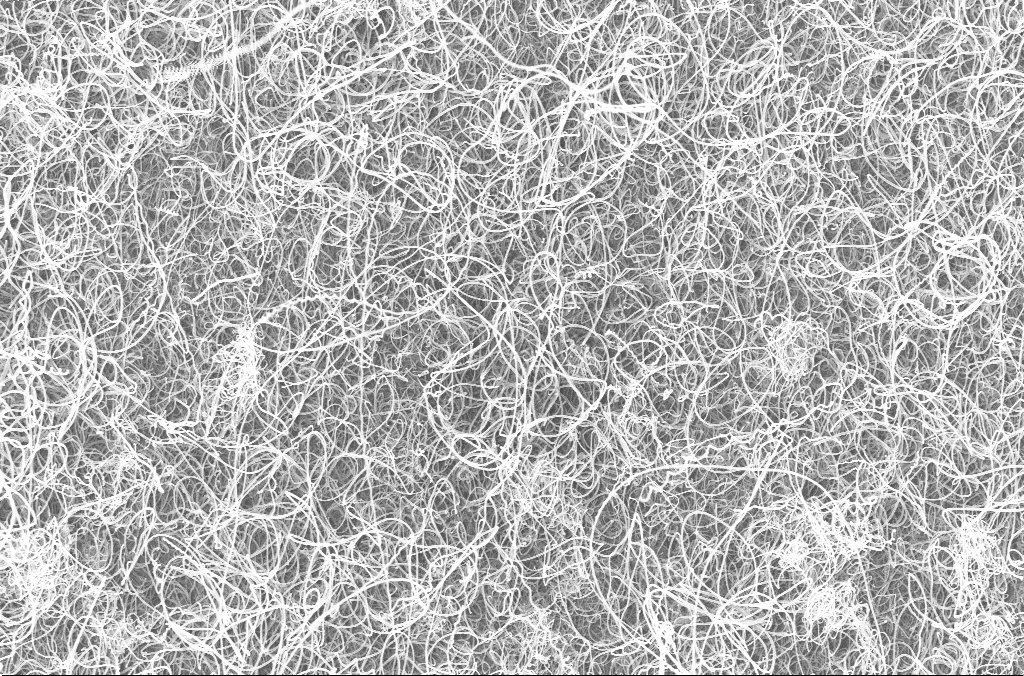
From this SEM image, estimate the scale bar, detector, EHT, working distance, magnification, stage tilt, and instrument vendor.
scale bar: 1000 nm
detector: InLens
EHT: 20 kV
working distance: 4.2 mm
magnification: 15 K X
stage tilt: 0°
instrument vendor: Zeiss